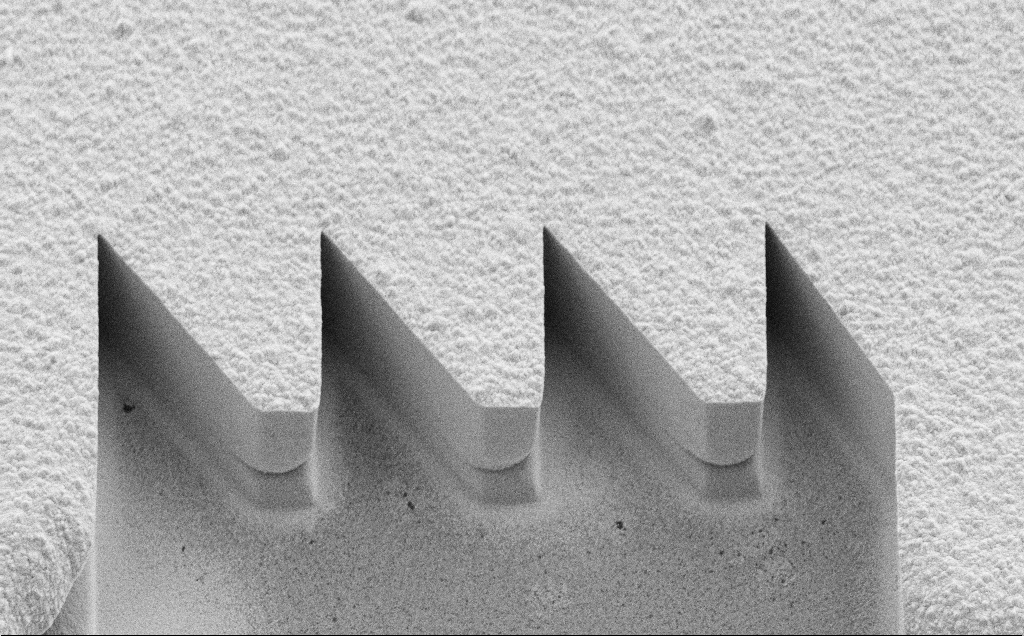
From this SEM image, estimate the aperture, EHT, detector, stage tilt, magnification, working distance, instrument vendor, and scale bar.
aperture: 30 µm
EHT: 10 kV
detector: SE2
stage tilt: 45°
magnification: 7.15 K X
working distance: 7 mm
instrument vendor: Zeiss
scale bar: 10000 nm